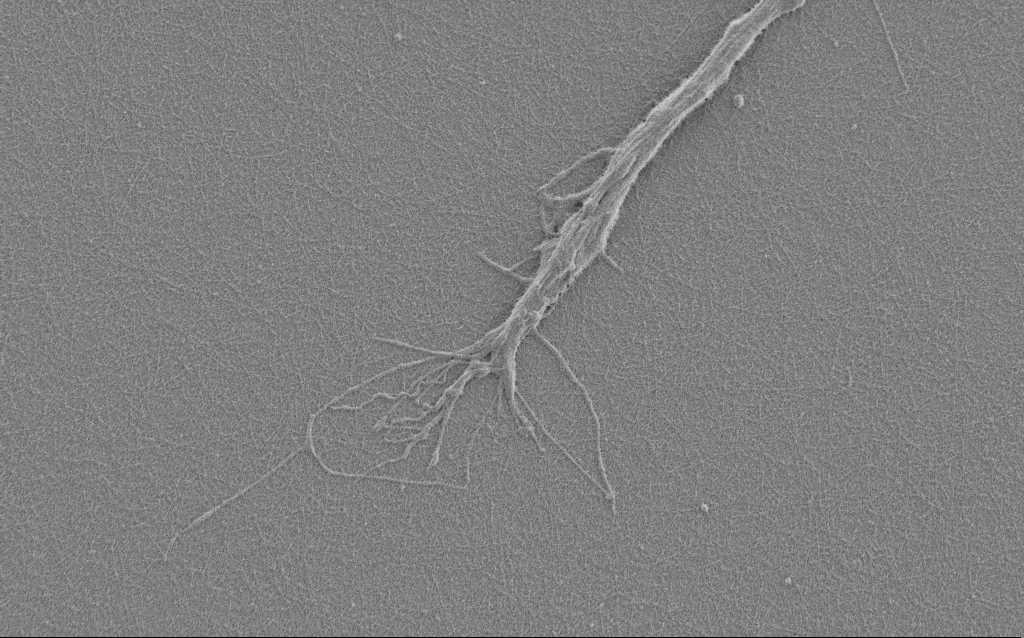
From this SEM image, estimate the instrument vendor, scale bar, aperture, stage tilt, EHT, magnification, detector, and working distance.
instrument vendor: Zeiss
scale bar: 2000 nm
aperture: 30 µm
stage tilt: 0°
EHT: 1 kV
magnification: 7.5 K X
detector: SE2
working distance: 6 mm